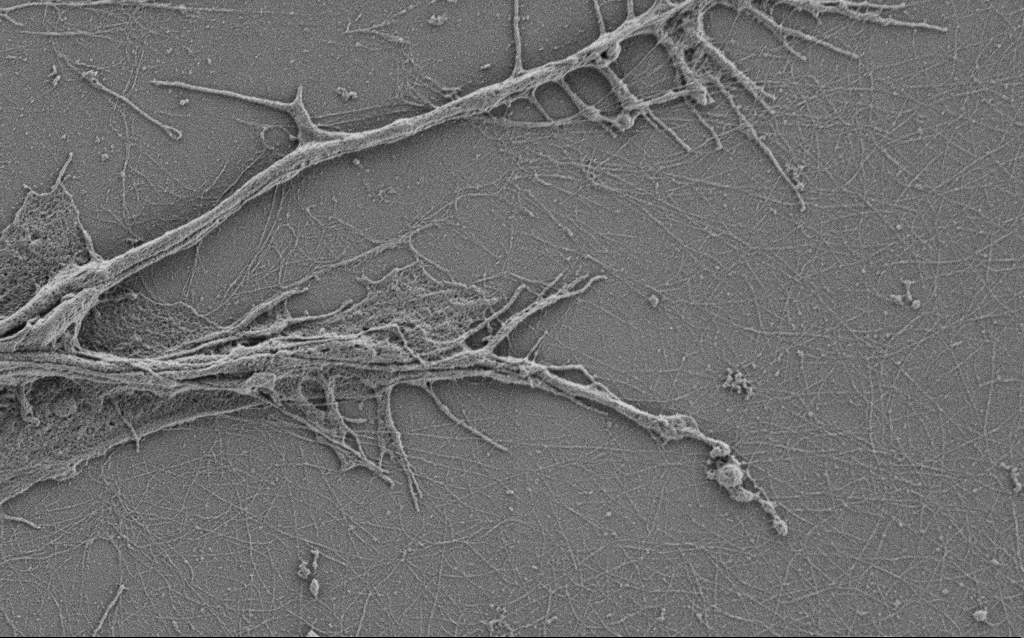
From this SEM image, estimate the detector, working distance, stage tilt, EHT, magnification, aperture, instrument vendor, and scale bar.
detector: SE2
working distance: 4 mm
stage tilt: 0°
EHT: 0.9 kV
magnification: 10 K X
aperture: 30 µm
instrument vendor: Zeiss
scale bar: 2000 nm